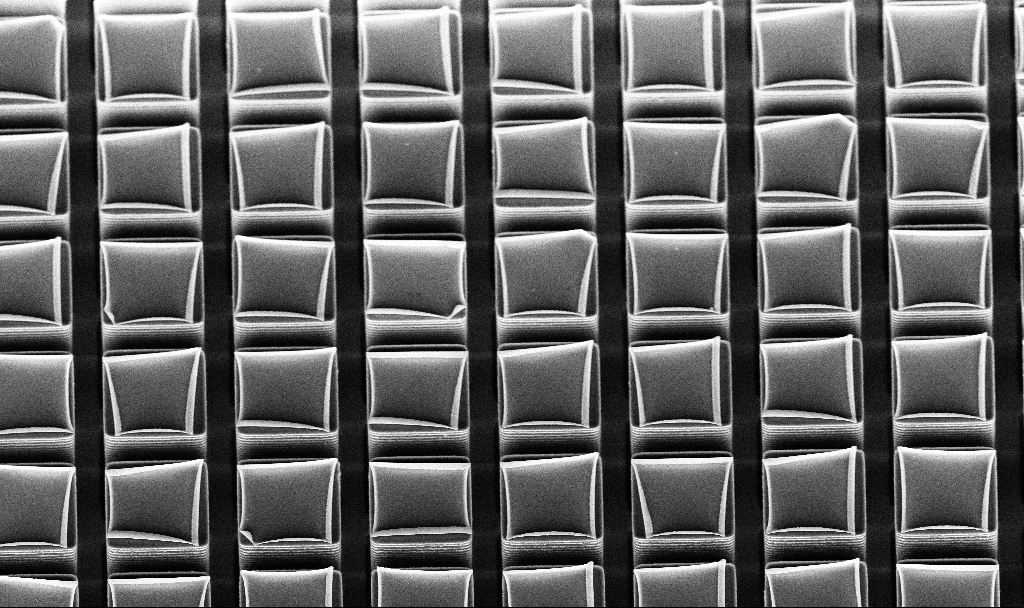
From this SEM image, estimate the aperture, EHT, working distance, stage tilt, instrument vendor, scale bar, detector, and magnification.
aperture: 30 µm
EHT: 5 kV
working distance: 9.5 mm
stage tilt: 30°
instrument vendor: Zeiss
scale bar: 10000 nm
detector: InLens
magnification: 4.82 K X